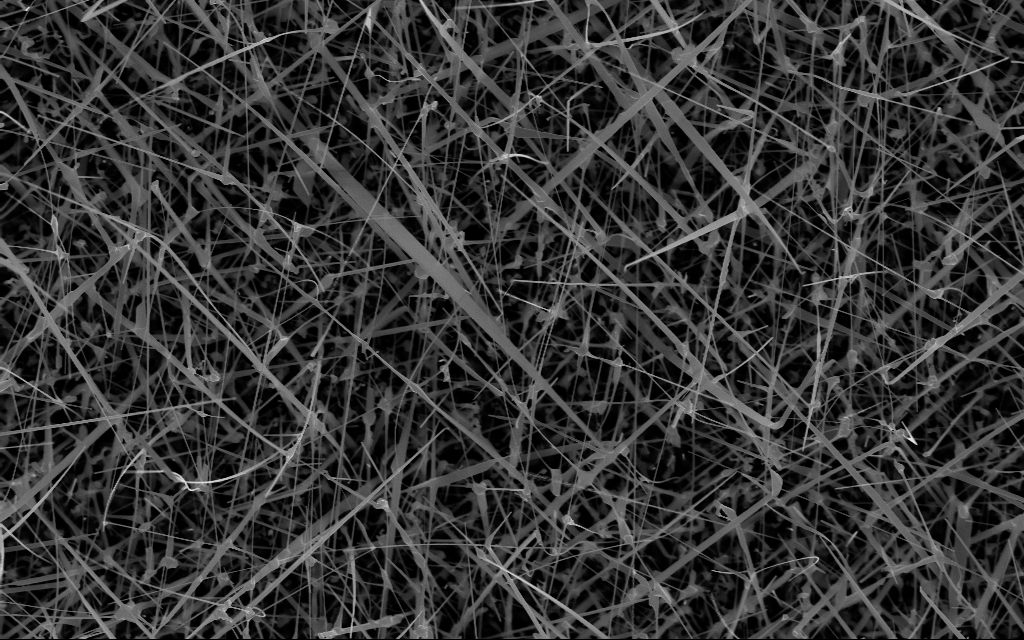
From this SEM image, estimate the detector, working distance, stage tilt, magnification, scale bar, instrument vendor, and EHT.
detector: InLens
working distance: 7 mm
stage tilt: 0°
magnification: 20 K X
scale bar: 2000 nm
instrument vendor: Zeiss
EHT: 10 kV